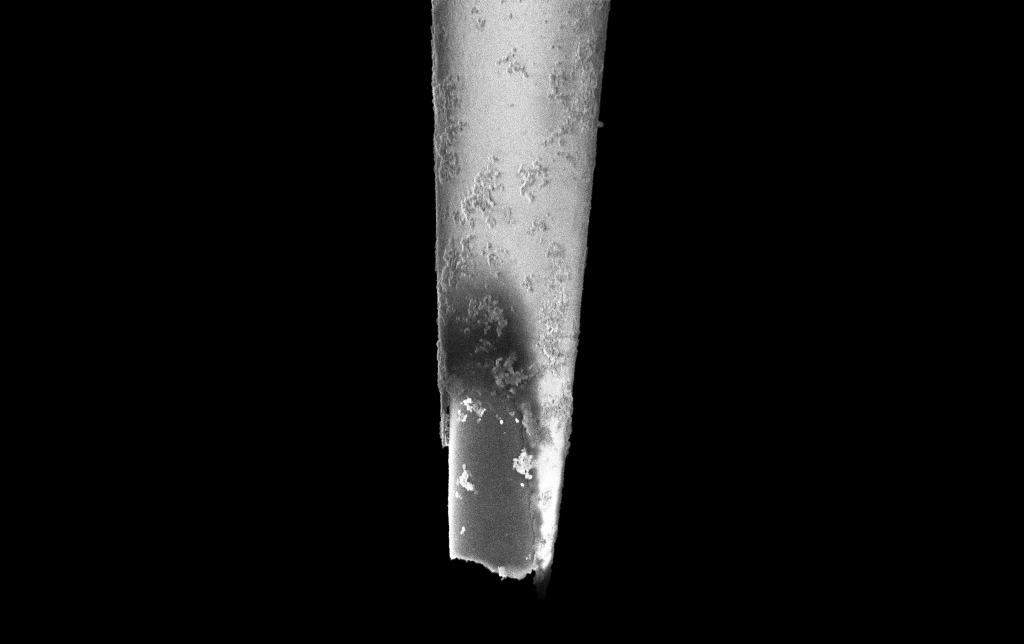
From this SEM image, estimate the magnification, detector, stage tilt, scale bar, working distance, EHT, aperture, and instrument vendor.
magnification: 25 K X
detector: InLens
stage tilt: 0°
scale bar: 2000 nm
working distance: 6 mm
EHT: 2 kV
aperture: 30 µm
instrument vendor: Zeiss